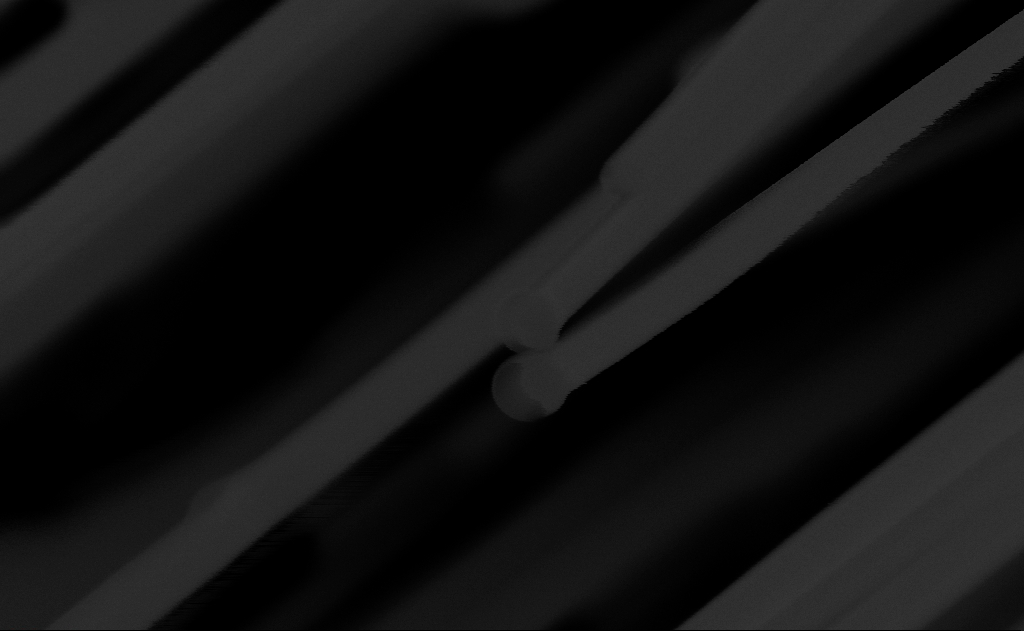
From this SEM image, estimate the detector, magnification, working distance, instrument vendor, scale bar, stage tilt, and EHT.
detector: InLens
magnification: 250 K X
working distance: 10 mm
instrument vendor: Zeiss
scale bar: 200 nm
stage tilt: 0°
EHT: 10 kV